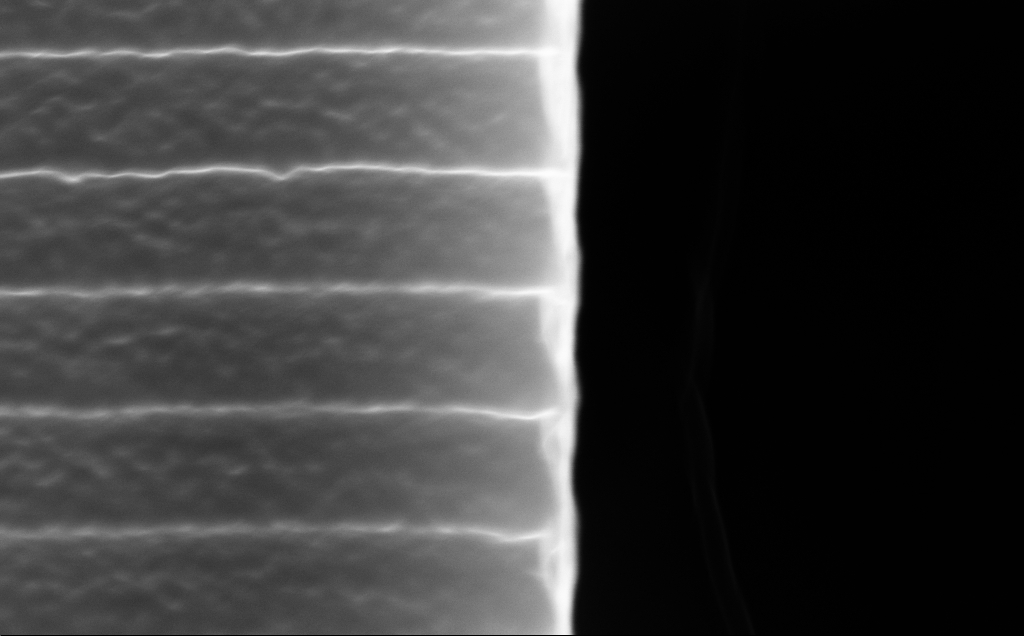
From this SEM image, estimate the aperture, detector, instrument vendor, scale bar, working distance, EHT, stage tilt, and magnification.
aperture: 30 µm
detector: InLens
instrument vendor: Zeiss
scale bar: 200 nm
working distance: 5 mm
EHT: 10 kV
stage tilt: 45°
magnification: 117.72 K X